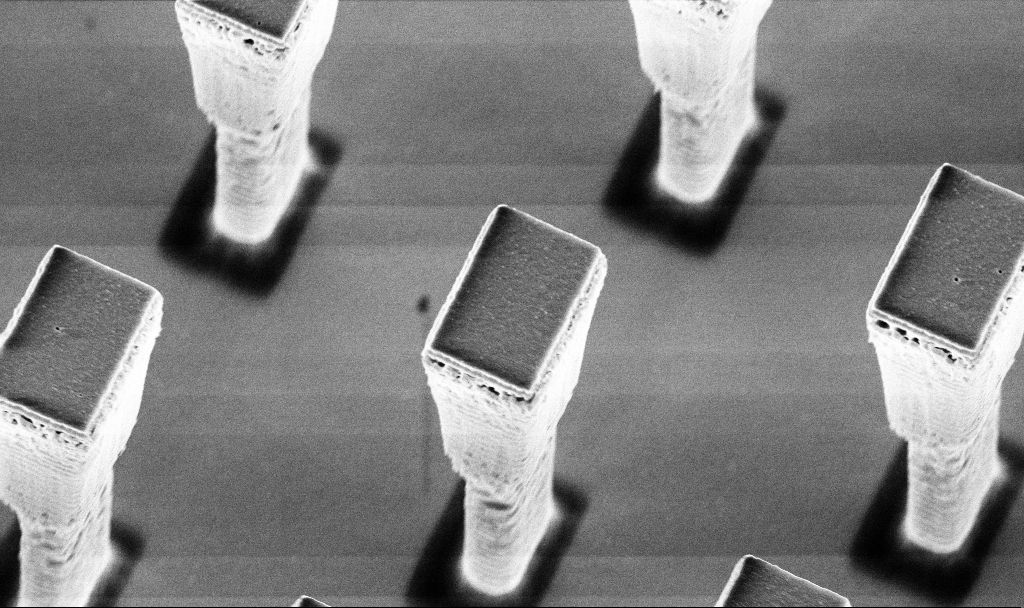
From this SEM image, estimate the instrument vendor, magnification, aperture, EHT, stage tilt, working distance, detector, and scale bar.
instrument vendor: Zeiss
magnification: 14.1 K X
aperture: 30 µm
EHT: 5 kV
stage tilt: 19.9°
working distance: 3.1 mm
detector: InLens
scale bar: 2000 nm